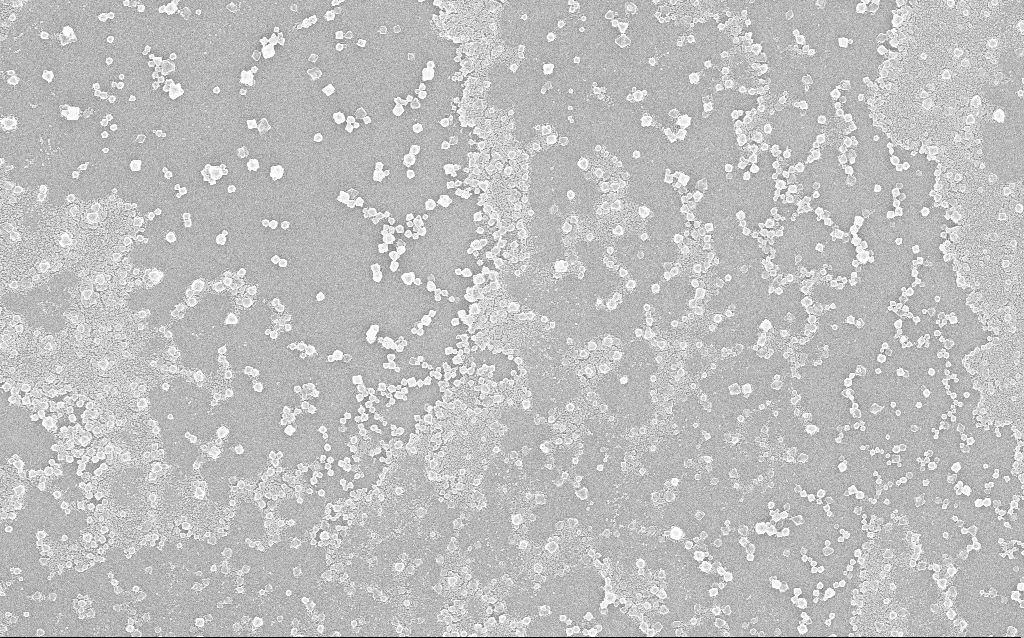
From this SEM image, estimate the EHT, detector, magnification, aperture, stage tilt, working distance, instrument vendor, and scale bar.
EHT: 20 kV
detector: InLens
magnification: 10 K X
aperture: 30 µm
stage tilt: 0°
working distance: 1.8 mm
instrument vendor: Zeiss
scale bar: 2000 nm